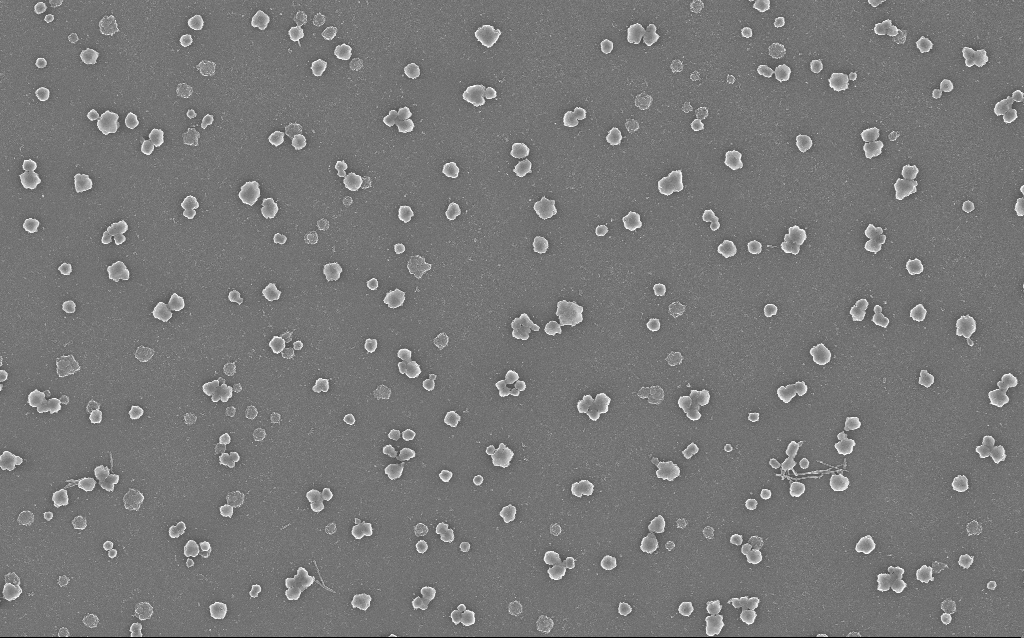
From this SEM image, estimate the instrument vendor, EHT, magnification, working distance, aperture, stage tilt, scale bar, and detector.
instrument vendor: Zeiss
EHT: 20 kV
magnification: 10 K X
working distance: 1.6 mm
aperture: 30 µm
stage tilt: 0°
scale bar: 2000 nm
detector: InLens